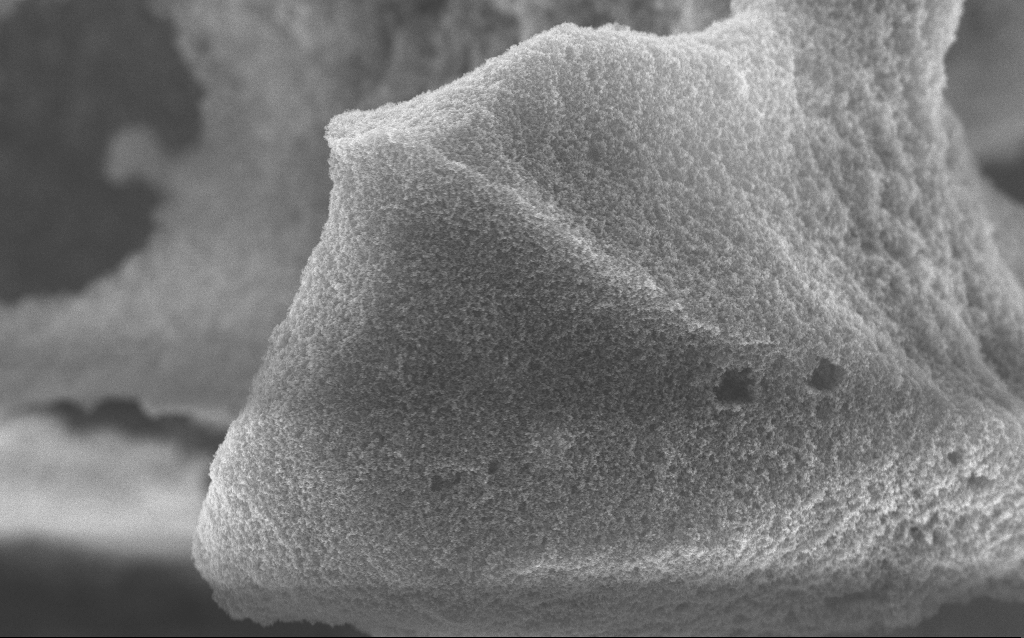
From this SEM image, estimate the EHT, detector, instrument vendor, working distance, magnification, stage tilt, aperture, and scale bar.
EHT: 10 kV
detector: InLens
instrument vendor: Zeiss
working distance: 2.7 mm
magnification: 20.87 K X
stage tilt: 0°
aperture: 30 µm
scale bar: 1000 nm